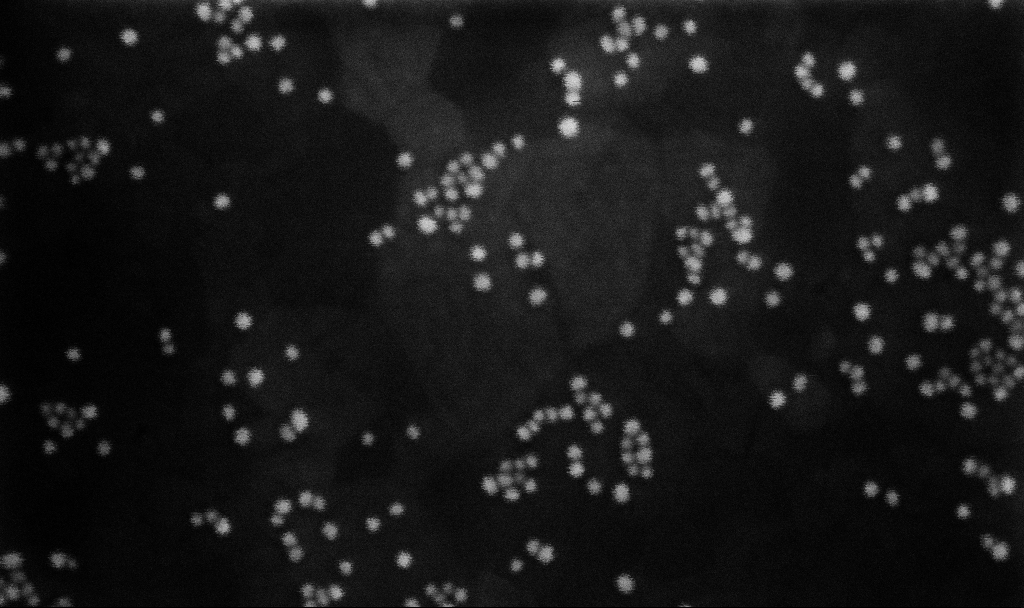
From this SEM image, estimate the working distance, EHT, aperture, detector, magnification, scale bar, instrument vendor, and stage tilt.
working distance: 7 mm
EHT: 5 kV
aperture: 30 µm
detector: InLens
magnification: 300 K X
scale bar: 200 nm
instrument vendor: Zeiss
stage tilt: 0°